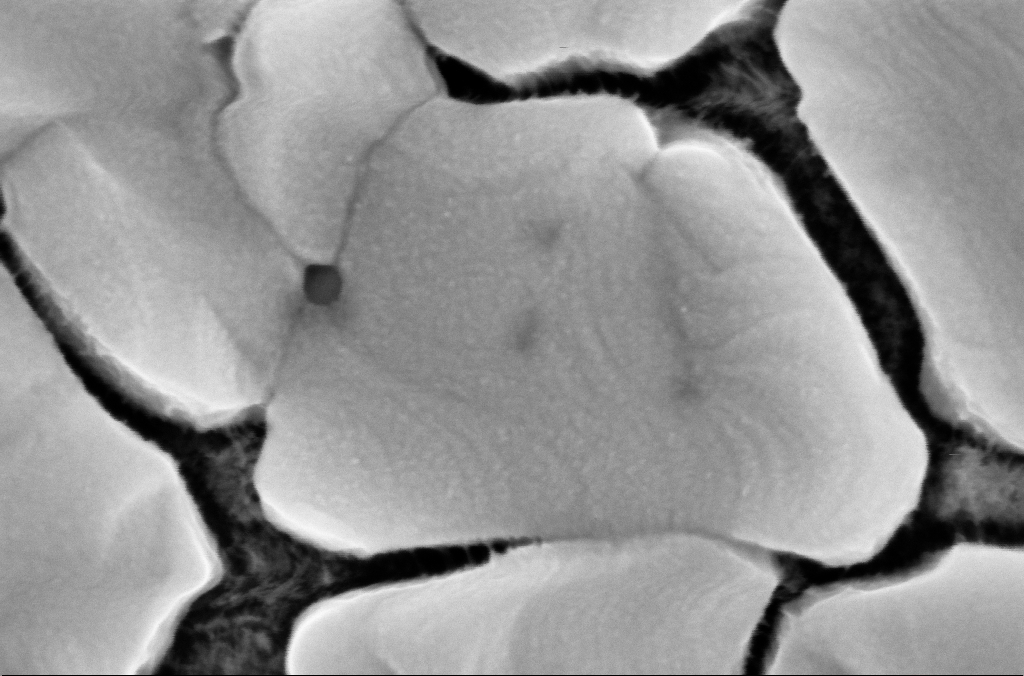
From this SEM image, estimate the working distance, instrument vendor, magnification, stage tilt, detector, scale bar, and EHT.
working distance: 3 mm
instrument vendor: Zeiss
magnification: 300 K X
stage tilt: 0°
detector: InLens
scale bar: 200 nm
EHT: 5 kV